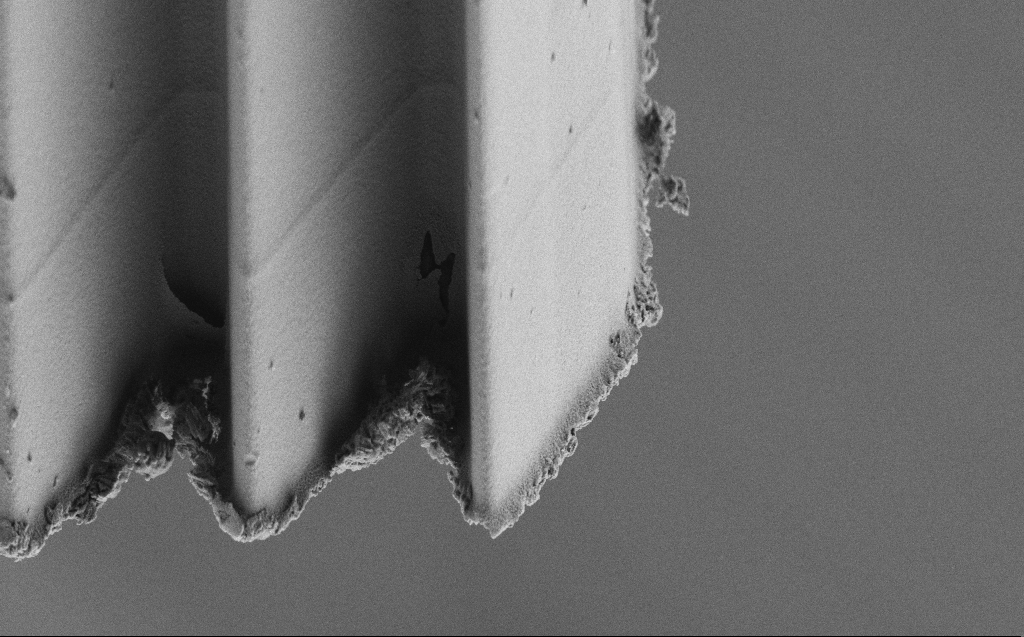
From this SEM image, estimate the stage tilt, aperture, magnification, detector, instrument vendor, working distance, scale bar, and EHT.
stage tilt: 45°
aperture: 30 µm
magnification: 7.76 K X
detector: SE2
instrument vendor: Zeiss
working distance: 4 mm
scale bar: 2000 nm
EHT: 3 kV